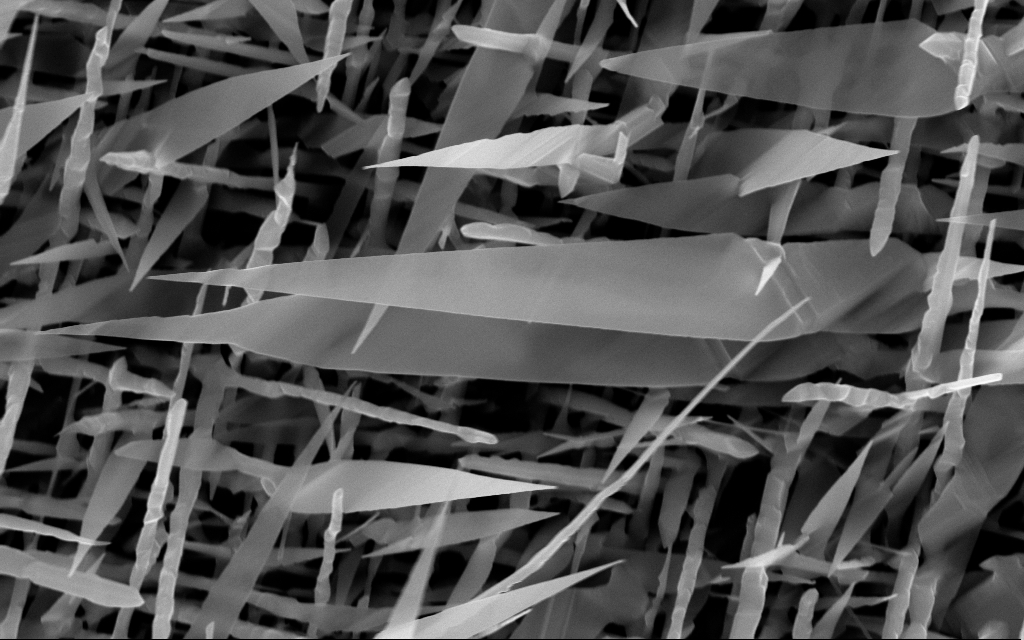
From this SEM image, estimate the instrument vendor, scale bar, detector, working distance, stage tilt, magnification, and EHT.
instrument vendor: Zeiss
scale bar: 1000 nm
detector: InLens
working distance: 7 mm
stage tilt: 0°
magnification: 40 K X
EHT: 10 kV